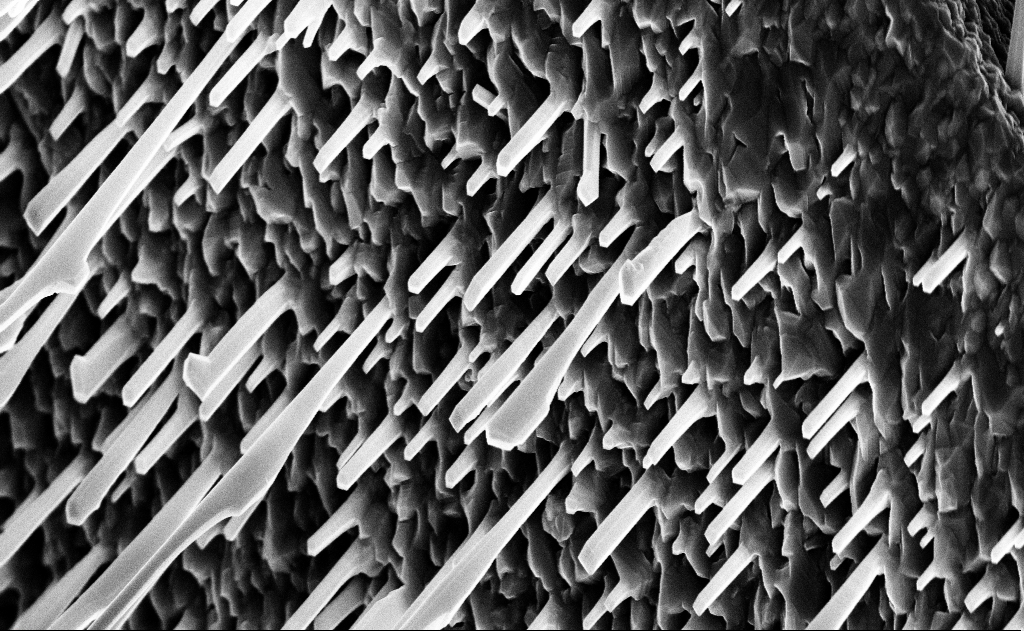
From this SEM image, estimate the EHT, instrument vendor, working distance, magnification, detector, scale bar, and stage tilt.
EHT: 10 kV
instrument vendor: Zeiss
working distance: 15 mm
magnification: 20 K X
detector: InLens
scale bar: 2000 nm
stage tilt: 0°